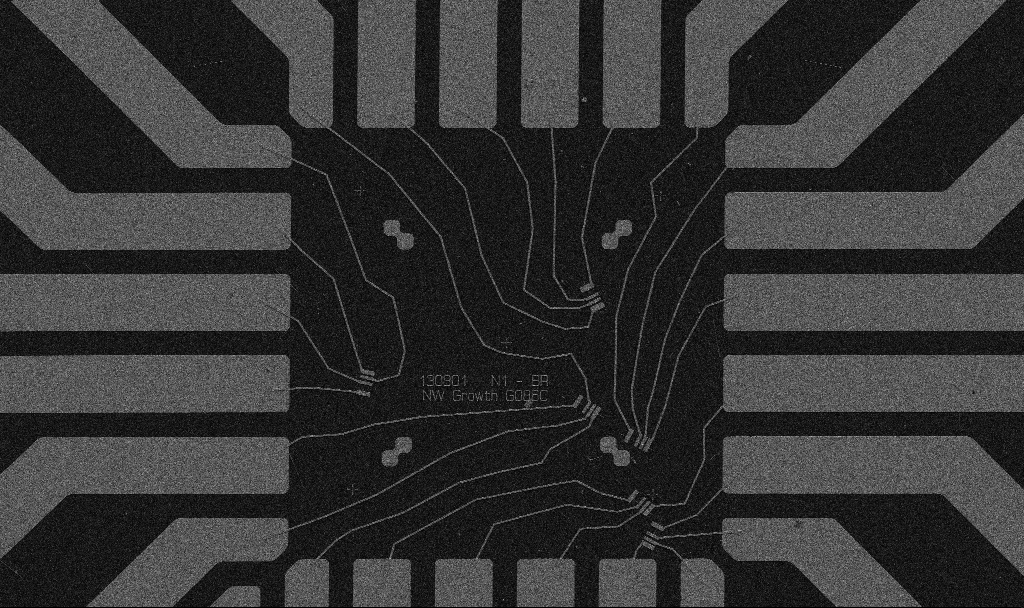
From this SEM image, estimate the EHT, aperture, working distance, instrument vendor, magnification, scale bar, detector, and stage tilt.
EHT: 5 kV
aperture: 30 µm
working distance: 10.7 mm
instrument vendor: Zeiss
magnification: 1 K X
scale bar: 20000 nm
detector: SE2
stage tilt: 0°